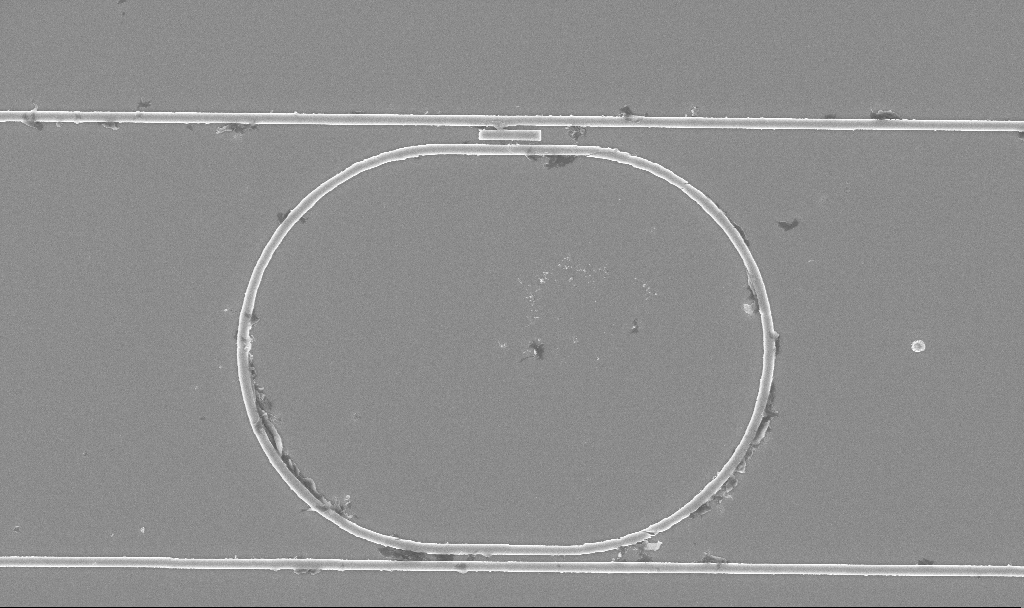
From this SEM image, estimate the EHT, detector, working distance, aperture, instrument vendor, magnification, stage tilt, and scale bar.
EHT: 5 kV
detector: InLens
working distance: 9.9 mm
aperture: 30 µm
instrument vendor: Zeiss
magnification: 7.59 K X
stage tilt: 0°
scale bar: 2000 nm